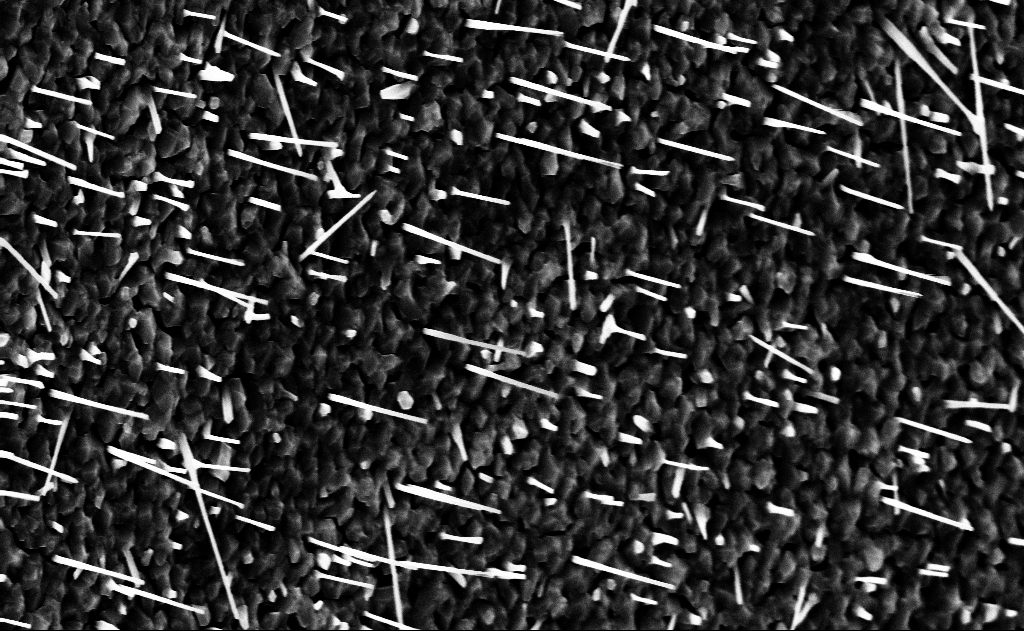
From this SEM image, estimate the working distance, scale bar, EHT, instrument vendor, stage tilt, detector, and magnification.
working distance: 14 mm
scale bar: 2000 nm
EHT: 10 kV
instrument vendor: Zeiss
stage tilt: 0°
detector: InLens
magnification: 20 K X